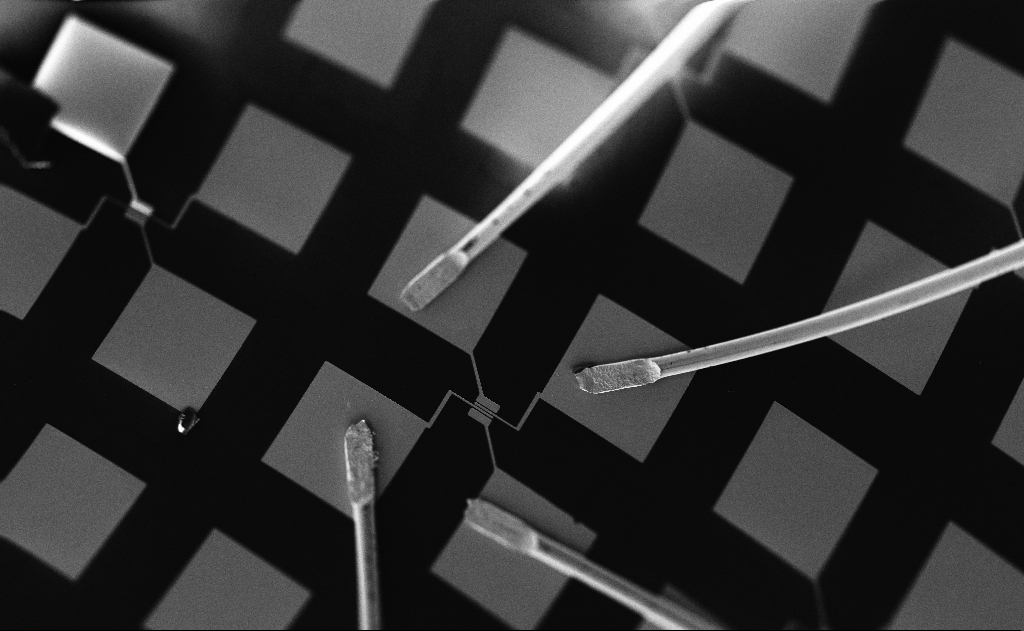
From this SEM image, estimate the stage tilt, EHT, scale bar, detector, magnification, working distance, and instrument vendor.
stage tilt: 0°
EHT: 5 kV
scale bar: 100000 nm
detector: InLens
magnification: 0.297 K X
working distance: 21 mm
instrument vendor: Zeiss